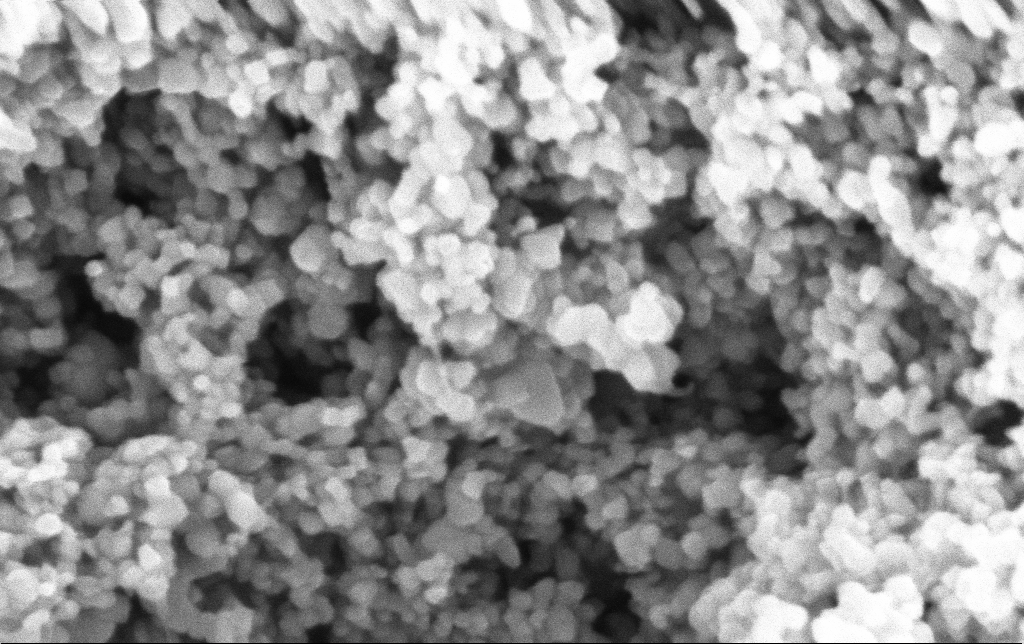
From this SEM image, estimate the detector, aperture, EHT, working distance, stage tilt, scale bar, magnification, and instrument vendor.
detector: InLens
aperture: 30 µm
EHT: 3 kV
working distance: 2.8 mm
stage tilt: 0°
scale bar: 200 nm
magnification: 305.95 K X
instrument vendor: Zeiss